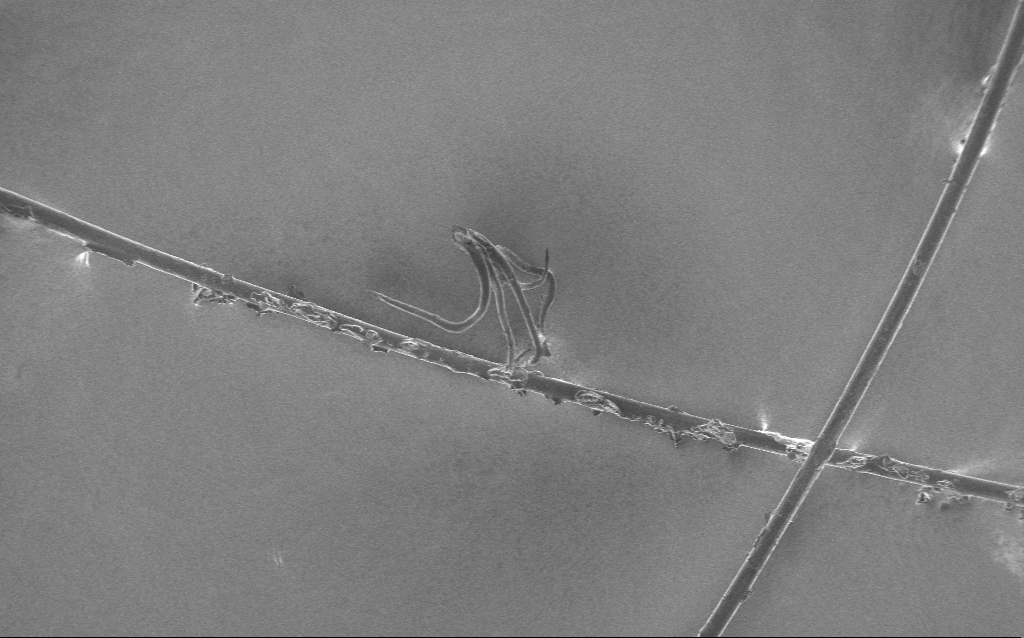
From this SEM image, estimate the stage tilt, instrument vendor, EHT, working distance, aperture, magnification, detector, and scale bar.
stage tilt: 0°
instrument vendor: Zeiss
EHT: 1 kV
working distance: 5 mm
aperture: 30 µm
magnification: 0.292 K X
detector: InLens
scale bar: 100000 nm